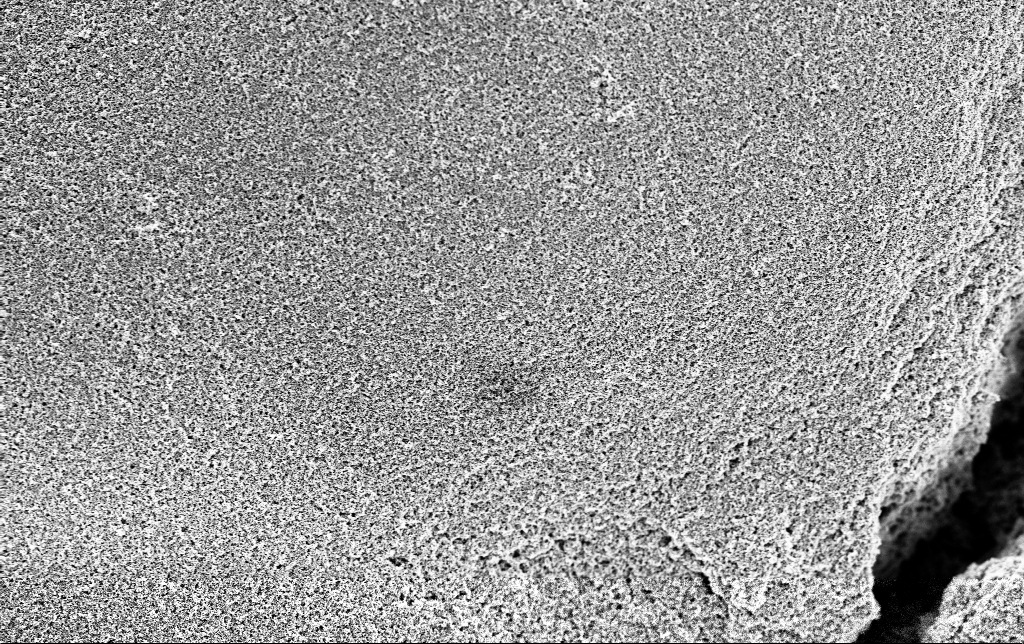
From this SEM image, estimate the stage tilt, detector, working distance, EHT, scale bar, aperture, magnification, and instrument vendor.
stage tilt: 0°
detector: InLens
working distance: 2.8 mm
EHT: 3 kV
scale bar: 2000 nm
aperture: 30 µm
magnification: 10 K X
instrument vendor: Zeiss